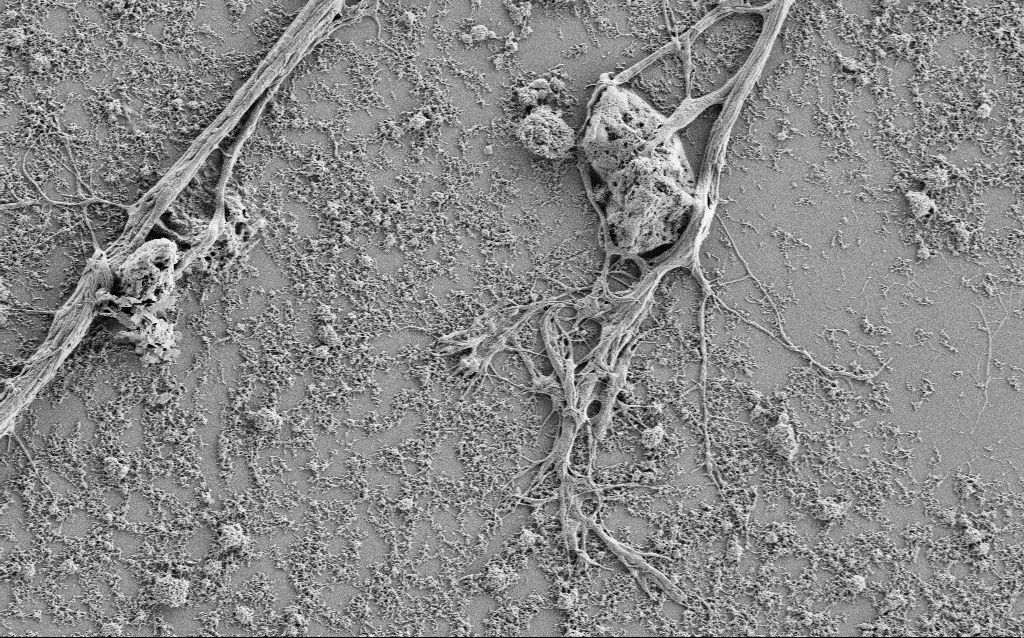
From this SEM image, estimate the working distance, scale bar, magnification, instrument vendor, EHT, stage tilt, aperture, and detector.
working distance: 4 mm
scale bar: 10000 nm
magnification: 5 K X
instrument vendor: Zeiss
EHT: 1 kV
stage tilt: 0°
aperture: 30 µm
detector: SE2